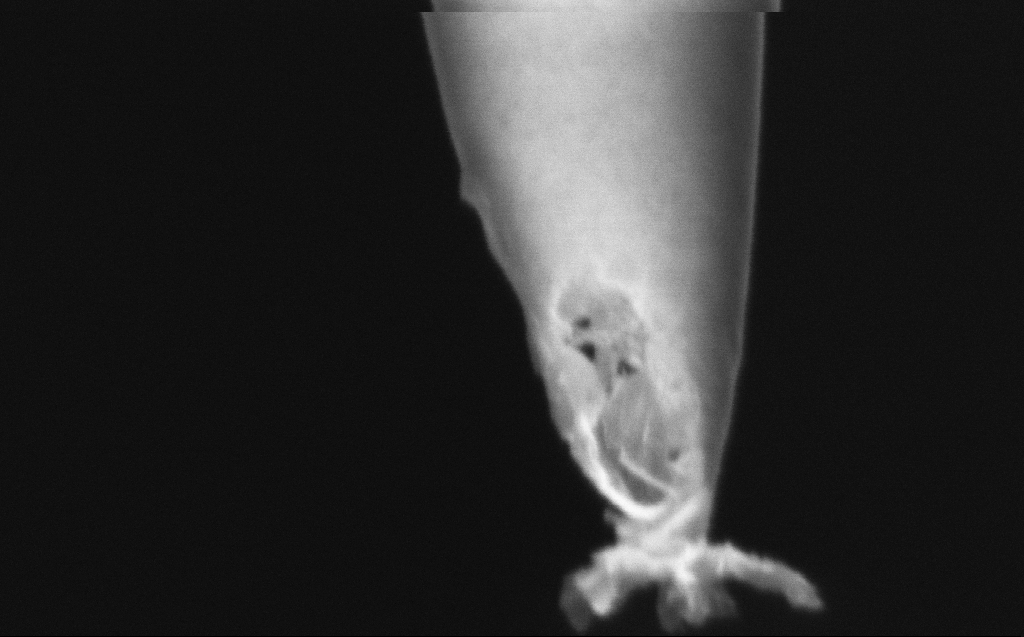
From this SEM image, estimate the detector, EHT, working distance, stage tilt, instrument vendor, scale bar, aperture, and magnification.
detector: InLens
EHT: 2 kV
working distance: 6 mm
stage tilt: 45°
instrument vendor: Zeiss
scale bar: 200 nm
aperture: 30 µm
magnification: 250 K X